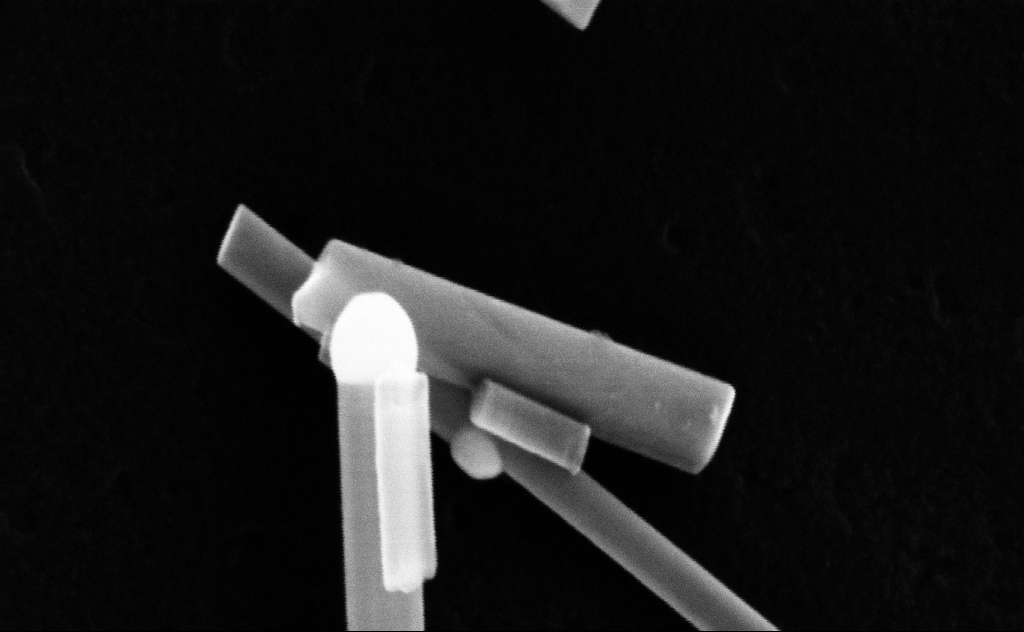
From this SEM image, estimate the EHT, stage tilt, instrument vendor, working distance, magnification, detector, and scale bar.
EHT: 20 kV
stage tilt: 0°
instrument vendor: Zeiss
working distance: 8 mm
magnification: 301.72 K X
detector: InLens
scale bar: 200 nm